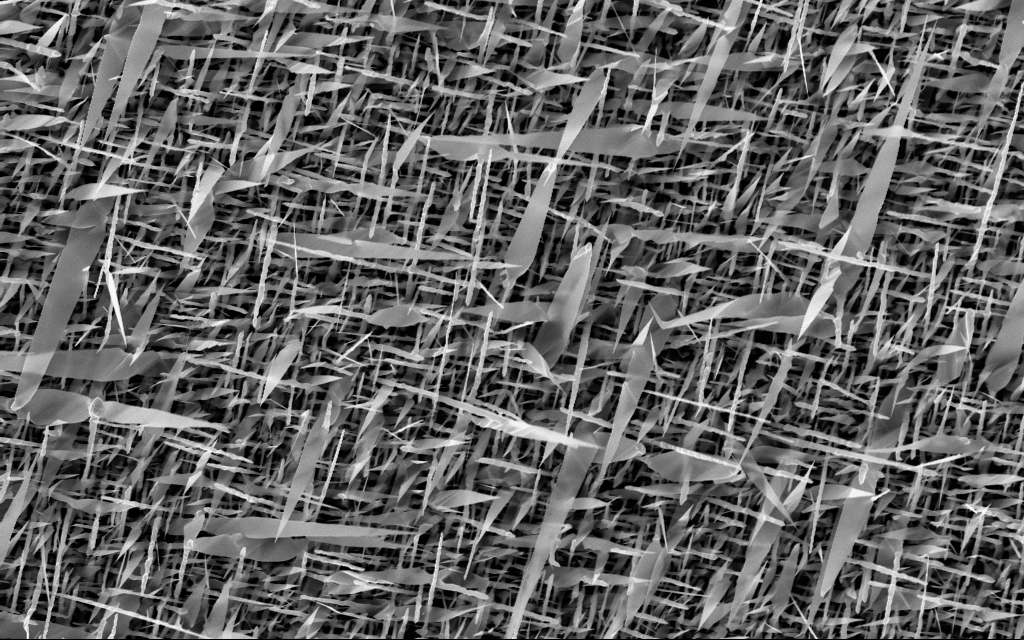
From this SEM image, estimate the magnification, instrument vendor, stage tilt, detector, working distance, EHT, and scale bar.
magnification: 10 K X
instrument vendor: Zeiss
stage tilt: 0°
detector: InLens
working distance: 7 mm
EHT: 10 kV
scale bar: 2000 nm